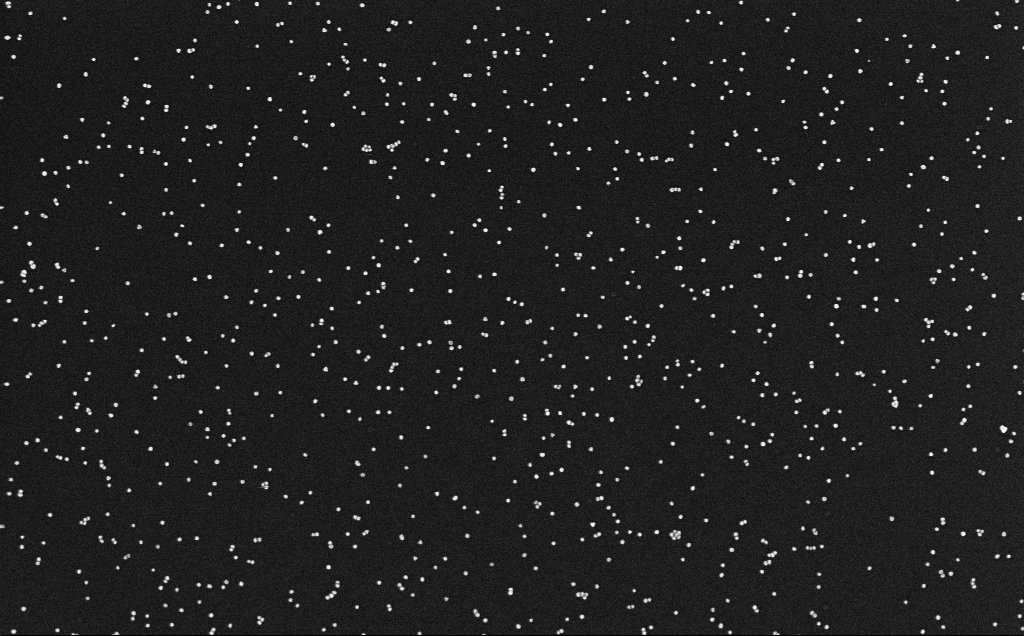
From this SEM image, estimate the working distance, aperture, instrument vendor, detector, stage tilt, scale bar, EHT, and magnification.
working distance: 3.1 mm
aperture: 30 µm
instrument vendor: Zeiss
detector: InLens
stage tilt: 0°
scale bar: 200 nm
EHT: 10 kV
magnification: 100 K X